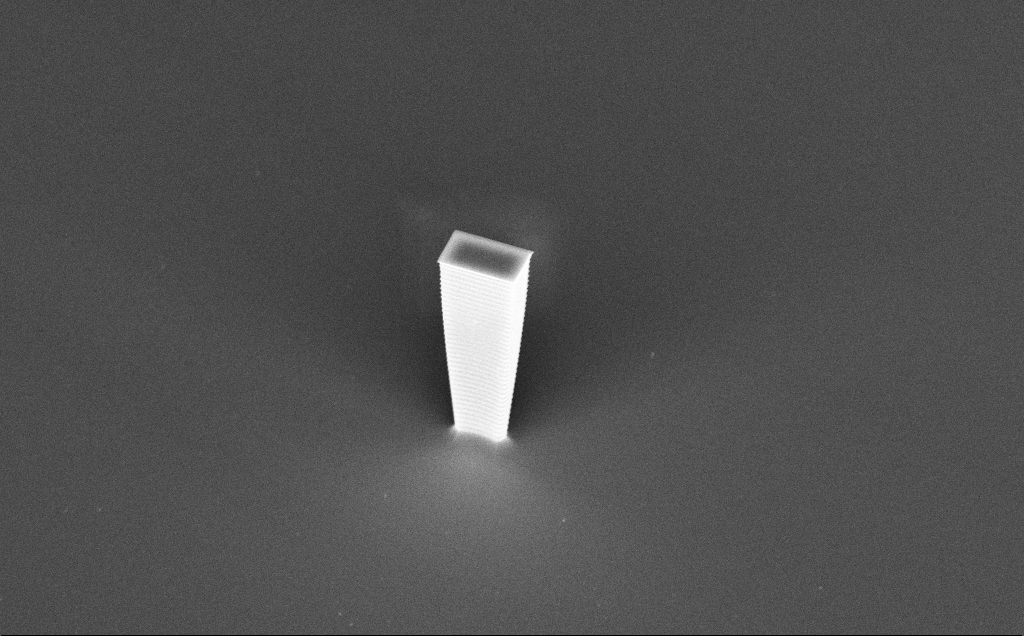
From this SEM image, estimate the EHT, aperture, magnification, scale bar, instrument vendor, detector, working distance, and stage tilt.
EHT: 10 kV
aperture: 30 µm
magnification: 6.4 K X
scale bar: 10000 nm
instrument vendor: Zeiss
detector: InLens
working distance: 7 mm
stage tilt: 45°